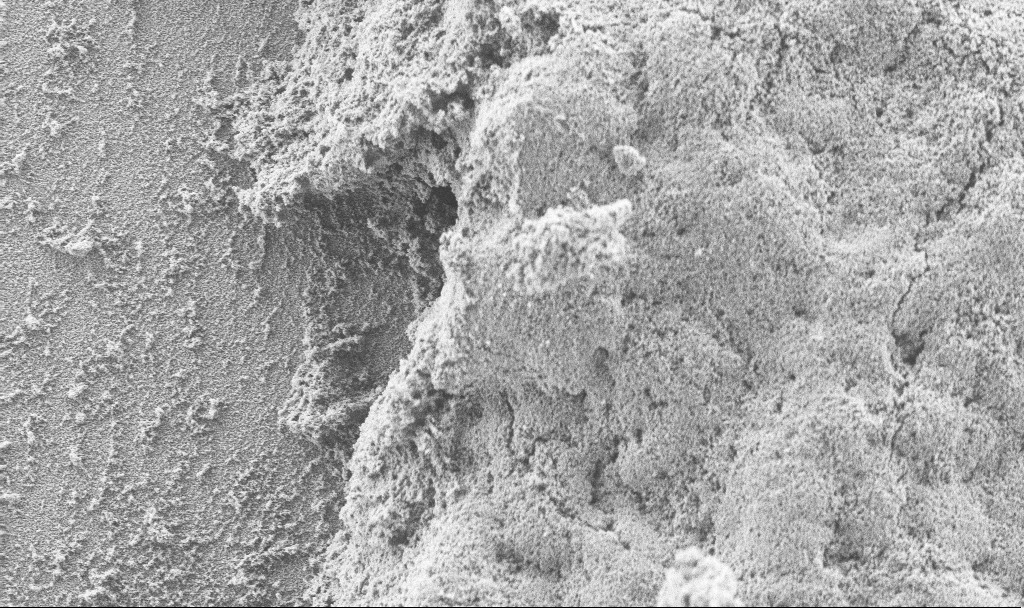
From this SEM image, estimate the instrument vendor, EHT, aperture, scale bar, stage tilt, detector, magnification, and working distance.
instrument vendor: Zeiss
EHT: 3 kV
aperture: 30 µm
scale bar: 2000 nm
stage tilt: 0°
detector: SE2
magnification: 7.29 K X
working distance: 2.7 mm